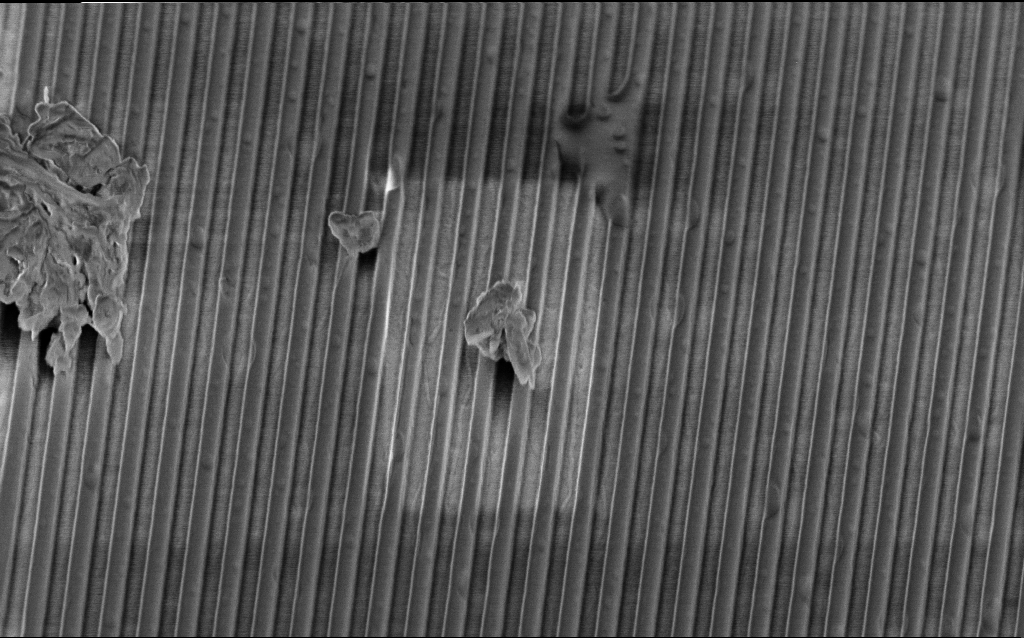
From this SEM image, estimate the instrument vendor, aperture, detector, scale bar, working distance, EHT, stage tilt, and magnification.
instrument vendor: Zeiss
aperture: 30 µm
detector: InLens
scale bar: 2000 nm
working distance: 3.8 mm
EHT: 2 kV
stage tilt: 45°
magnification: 27.97 K X